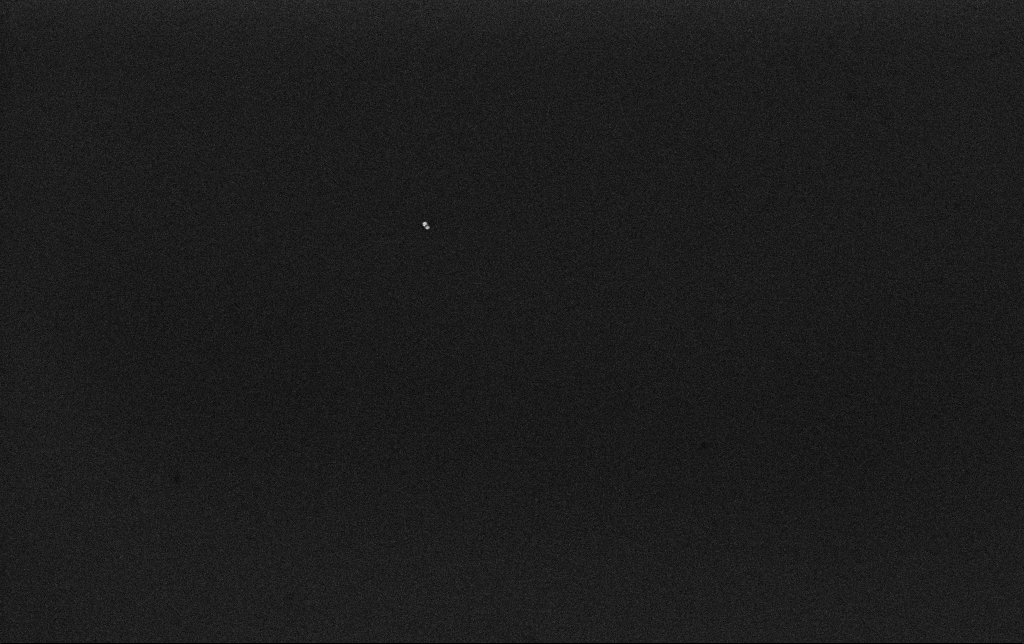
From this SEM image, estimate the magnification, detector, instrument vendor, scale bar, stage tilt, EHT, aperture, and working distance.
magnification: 100 K X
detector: InLens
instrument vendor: Zeiss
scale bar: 200 nm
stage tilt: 0°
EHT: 10 kV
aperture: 30 µm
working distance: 3.2 mm